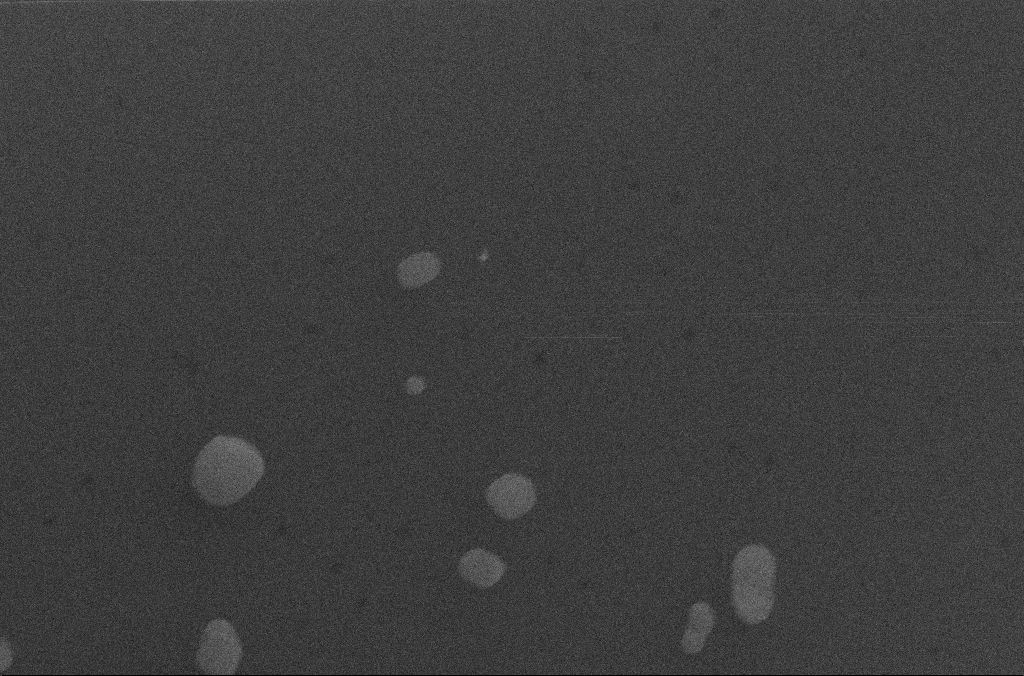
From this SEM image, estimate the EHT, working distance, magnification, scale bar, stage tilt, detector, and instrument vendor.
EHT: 20 kV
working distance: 2.8 mm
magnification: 15 K X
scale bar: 2000 nm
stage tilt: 20°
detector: SE2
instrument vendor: Zeiss